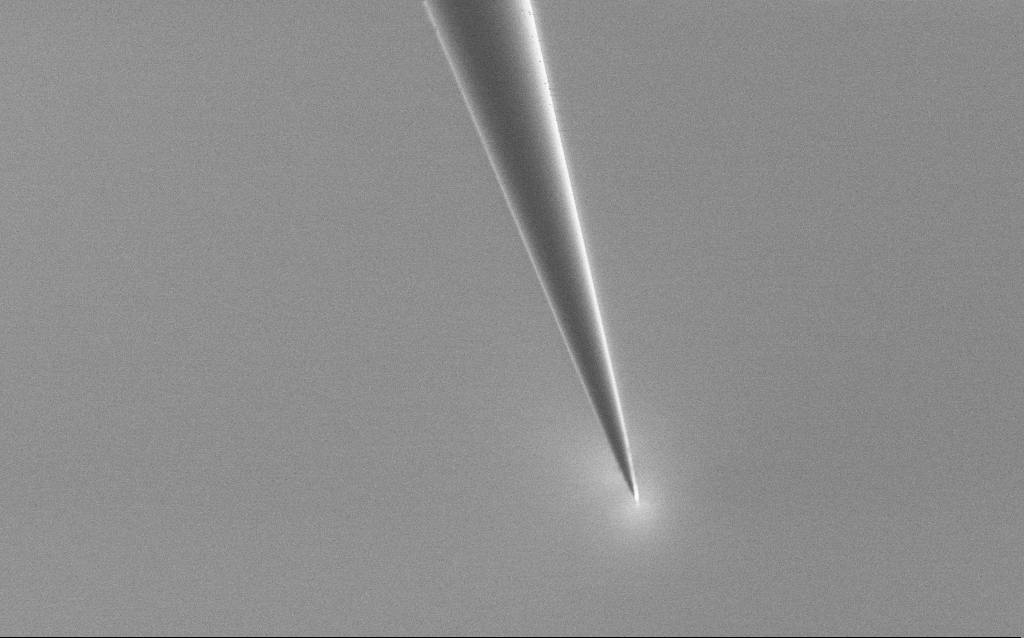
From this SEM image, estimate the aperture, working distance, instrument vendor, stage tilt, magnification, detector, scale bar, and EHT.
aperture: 30 µm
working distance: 6 mm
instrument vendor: Zeiss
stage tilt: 45°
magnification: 5 K X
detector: SE2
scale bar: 10000 nm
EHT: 1 kV